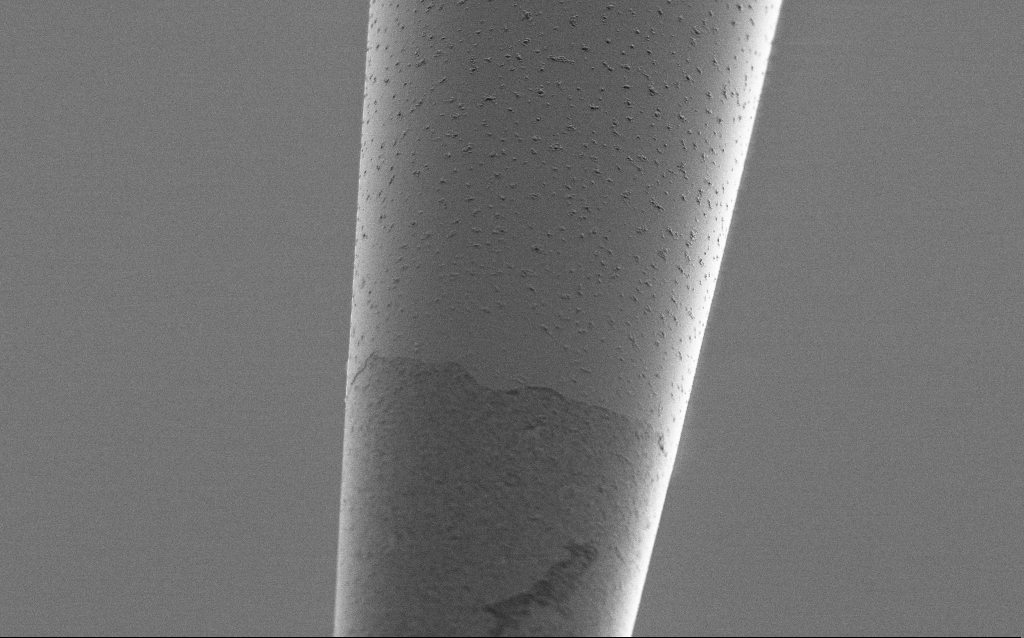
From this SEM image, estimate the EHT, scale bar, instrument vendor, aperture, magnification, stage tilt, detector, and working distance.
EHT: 1 kV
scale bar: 2000 nm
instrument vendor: Zeiss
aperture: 30 µm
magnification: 10 K X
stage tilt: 45°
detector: SE2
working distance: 6 mm